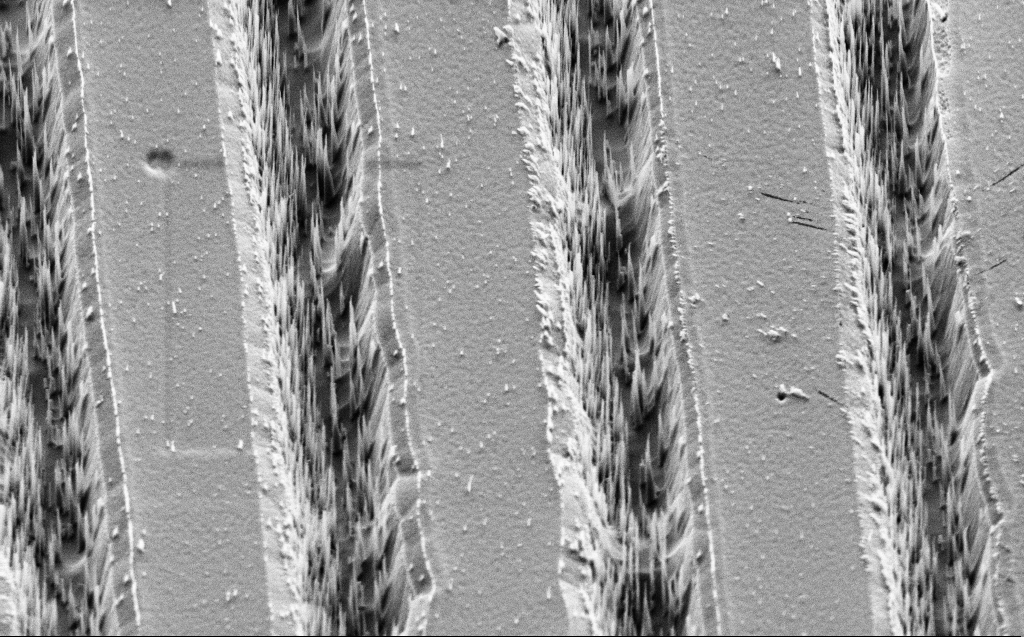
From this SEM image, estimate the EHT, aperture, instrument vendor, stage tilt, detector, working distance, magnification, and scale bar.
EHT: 3 kV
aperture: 30 µm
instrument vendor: Zeiss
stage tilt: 45°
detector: SE2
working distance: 8 mm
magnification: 10.77 K X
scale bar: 2000 nm